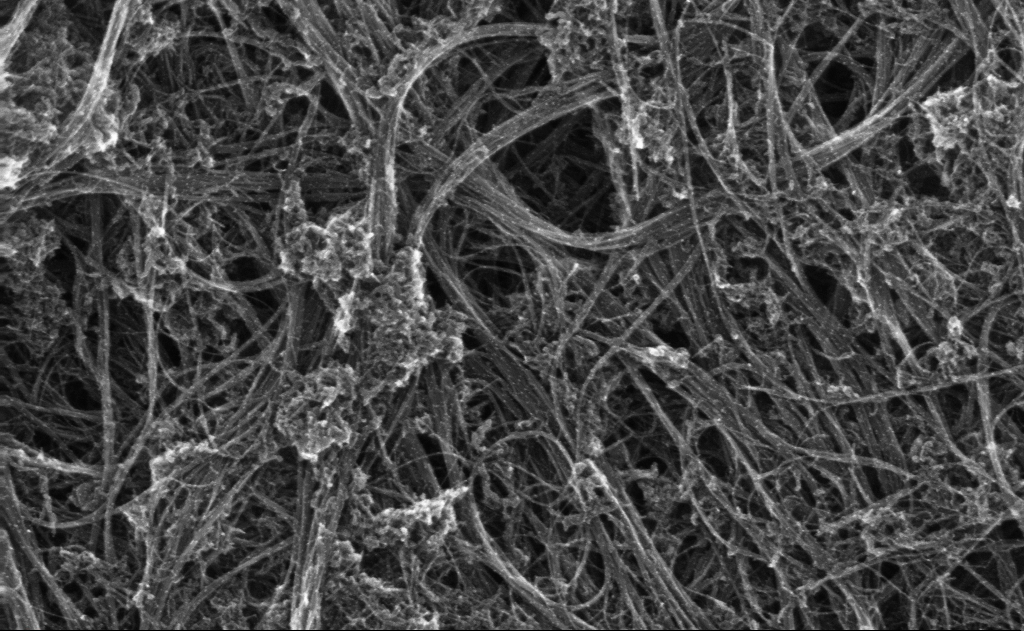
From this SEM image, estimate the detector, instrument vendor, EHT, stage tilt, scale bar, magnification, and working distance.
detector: InLens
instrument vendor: Zeiss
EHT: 10 kV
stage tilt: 0°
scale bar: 100 nm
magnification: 173.01 K X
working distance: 3 mm